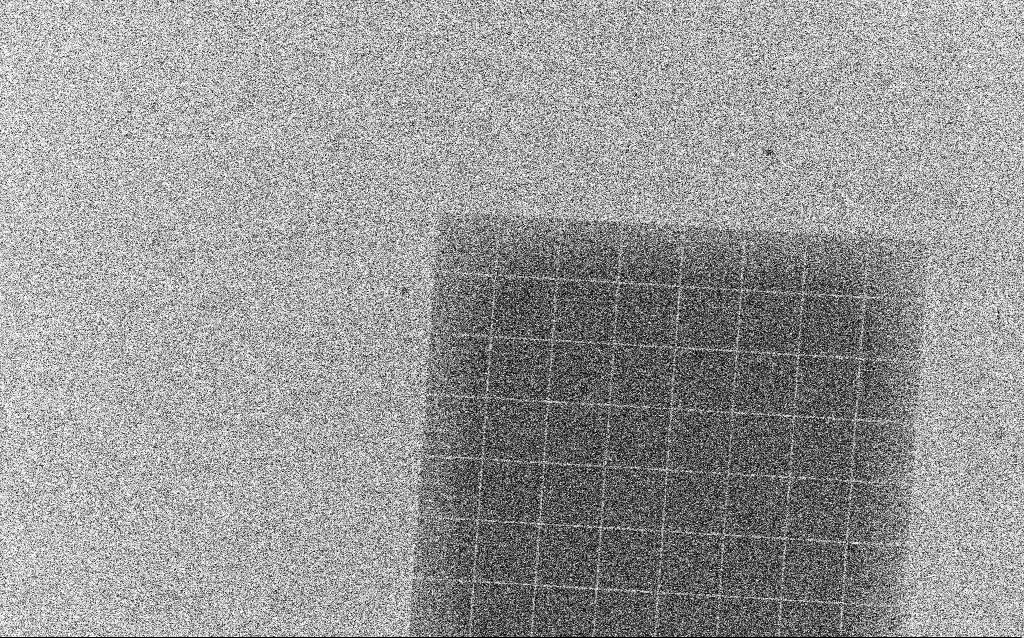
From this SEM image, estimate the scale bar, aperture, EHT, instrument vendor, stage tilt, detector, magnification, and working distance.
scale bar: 20000 nm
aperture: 30 µm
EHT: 3 kV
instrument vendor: Zeiss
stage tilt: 0°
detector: InLens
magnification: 1.01 K X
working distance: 4.4 mm